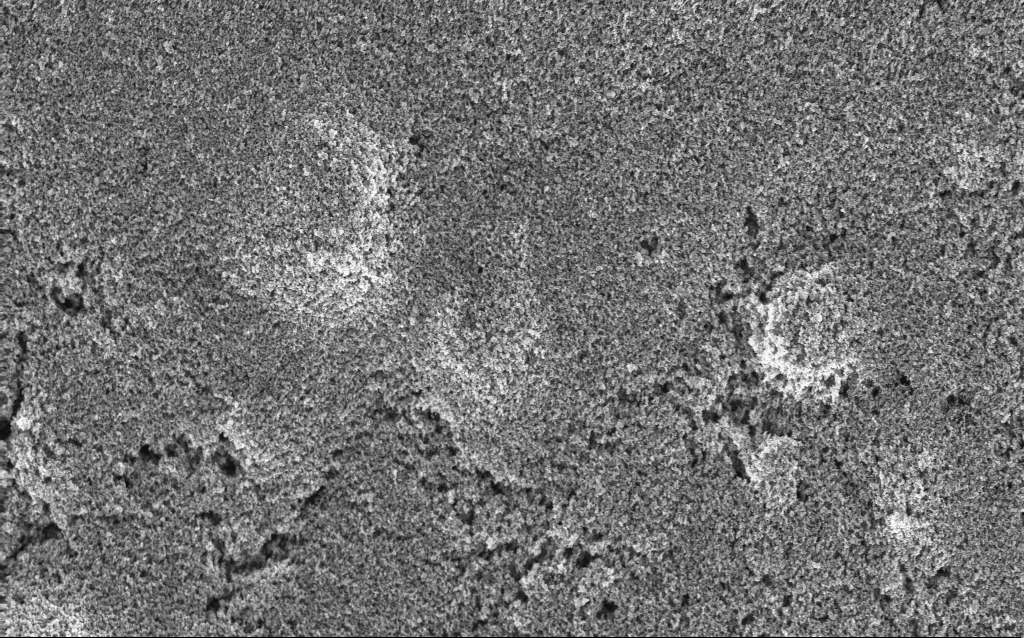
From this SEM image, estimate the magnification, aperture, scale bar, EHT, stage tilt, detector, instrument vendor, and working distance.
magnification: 20.87 K X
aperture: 30 µm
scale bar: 1000 nm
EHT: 5 kV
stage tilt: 0°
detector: InLens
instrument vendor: Zeiss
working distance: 2.7 mm